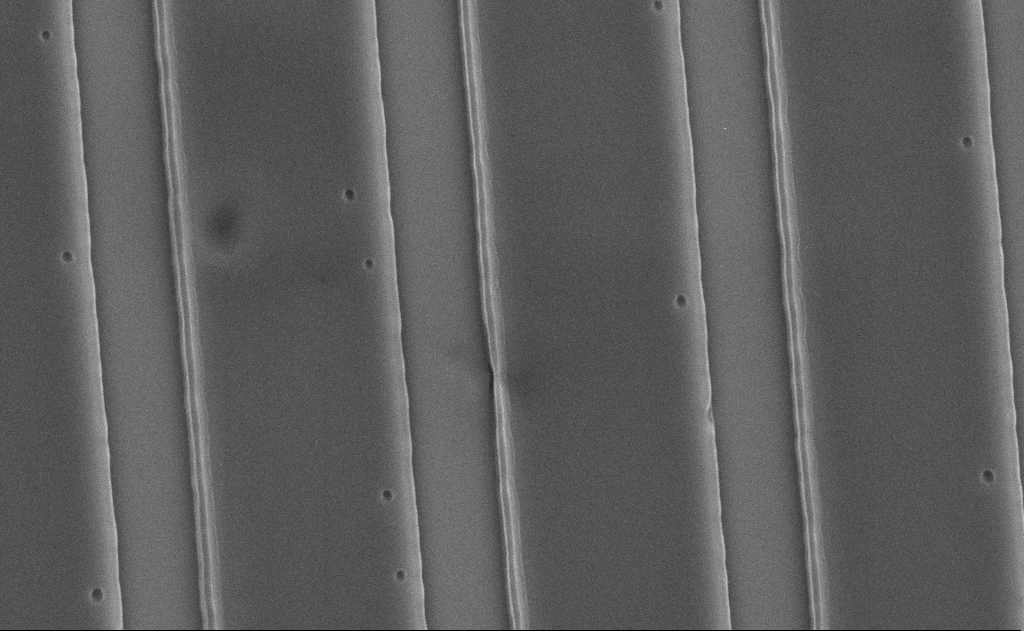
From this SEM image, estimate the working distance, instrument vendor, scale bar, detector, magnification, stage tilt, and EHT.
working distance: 12 mm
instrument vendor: Zeiss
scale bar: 1000 nm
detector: SE2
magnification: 27.5 K X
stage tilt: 0°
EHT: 5 kV